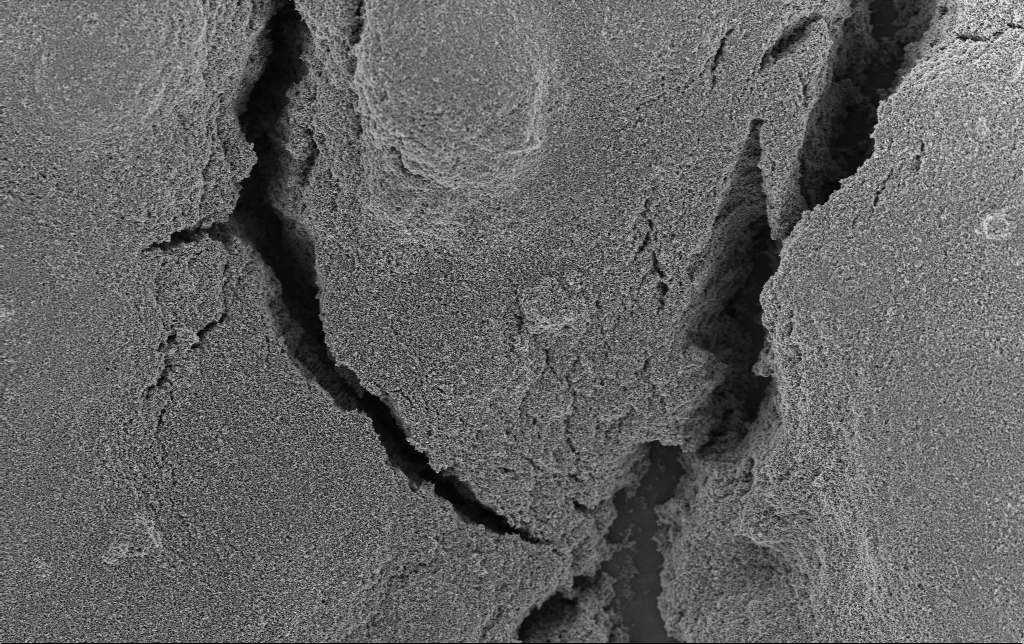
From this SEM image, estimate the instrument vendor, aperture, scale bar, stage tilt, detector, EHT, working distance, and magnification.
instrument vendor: Zeiss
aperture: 30 µm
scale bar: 10000 nm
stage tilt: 0°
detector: InLens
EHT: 3 kV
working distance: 2.8 mm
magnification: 5 K X